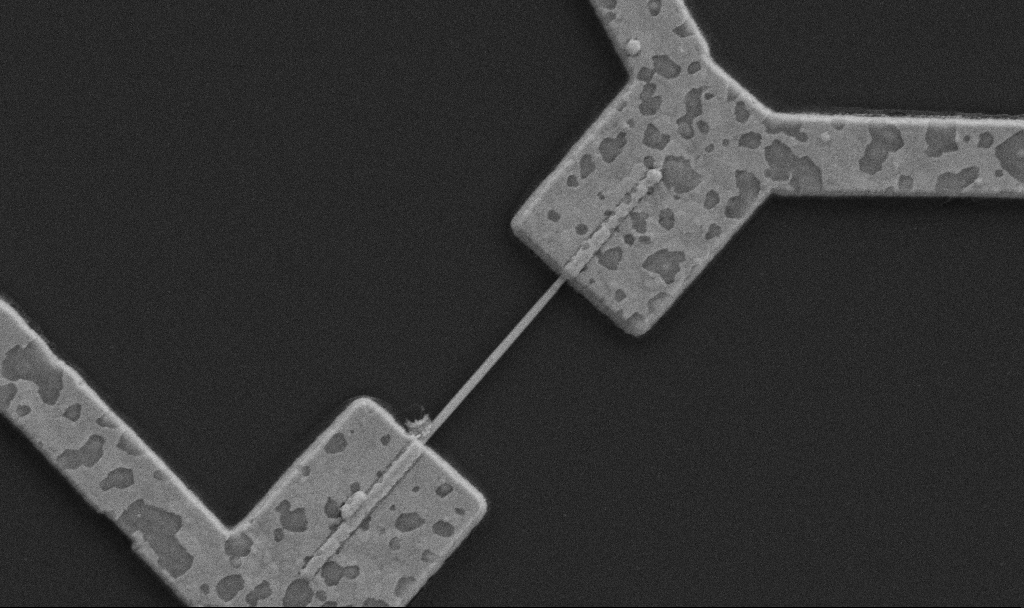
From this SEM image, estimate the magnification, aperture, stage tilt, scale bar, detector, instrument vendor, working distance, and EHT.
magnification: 30 K X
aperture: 30 µm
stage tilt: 0°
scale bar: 1000 nm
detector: SE2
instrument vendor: Zeiss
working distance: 10.7 mm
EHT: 5 kV